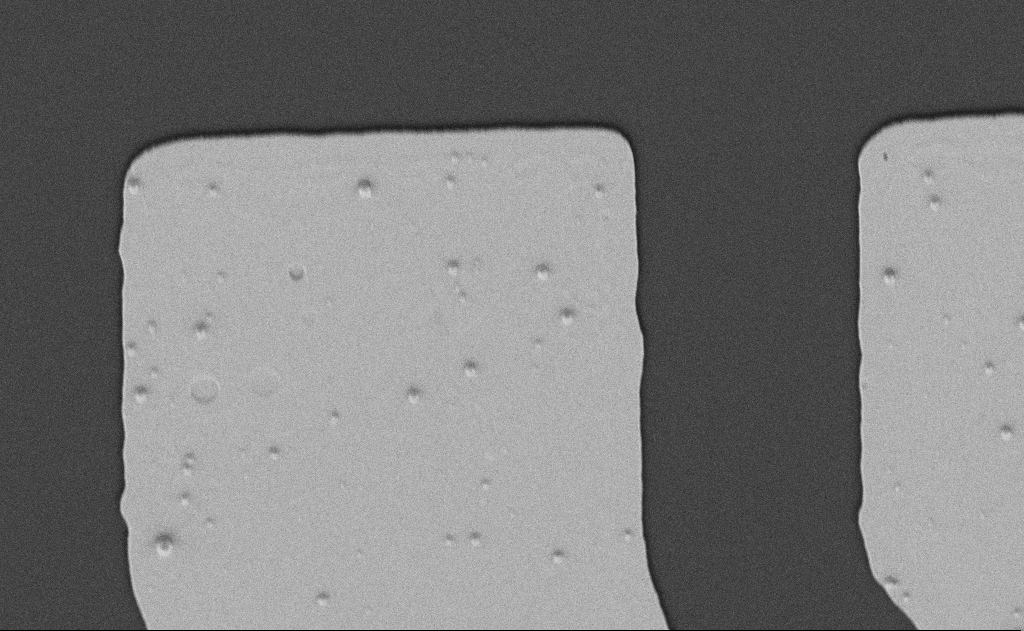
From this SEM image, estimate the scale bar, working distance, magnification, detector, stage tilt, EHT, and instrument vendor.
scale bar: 2000 nm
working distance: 6 mm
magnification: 9.06 K X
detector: SE2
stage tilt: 30.1°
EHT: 5 kV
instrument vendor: Zeiss